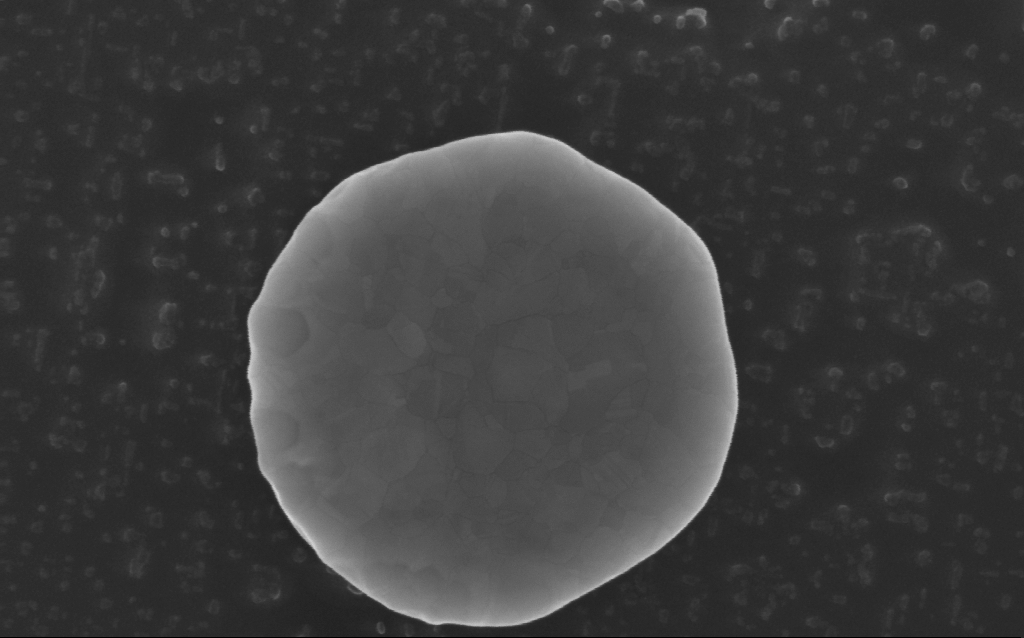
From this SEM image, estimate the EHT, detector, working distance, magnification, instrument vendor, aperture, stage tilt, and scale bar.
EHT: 10 kV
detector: InLens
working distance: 5 mm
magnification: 195.65 K X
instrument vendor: Zeiss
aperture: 30 µm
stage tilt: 0°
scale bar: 100 nm